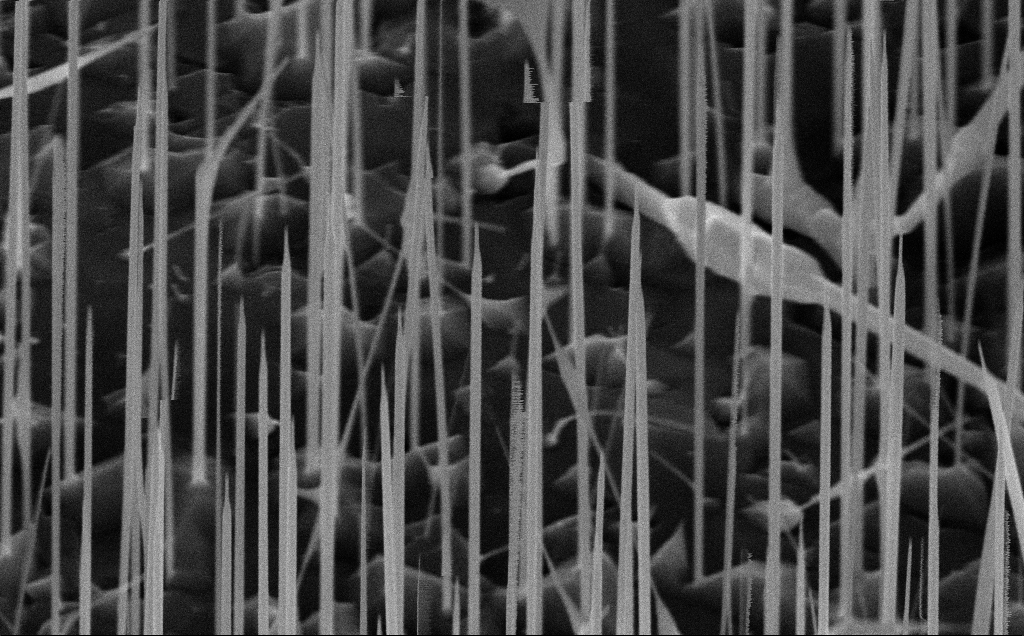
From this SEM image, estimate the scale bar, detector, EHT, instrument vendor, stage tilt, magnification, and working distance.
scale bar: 1000 nm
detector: InLens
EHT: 10 kV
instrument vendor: Zeiss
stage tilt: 45°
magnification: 61.05 K X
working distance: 8 mm